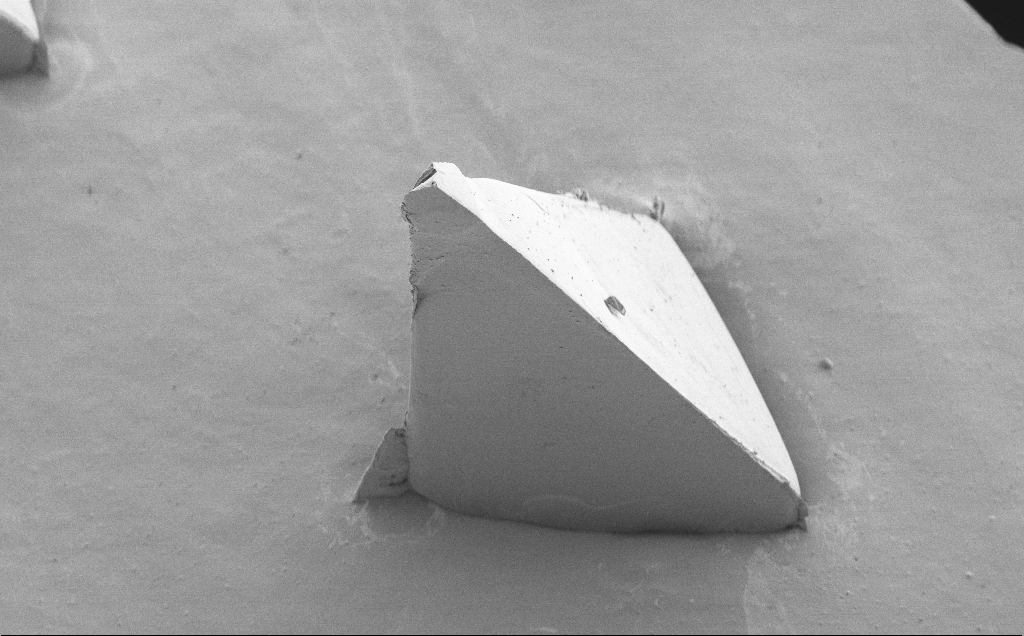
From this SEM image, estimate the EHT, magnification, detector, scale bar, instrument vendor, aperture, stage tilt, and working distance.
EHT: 5 kV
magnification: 0.236 K X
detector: SE2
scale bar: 200000 nm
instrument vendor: Zeiss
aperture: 30 µm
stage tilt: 40°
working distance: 8 mm